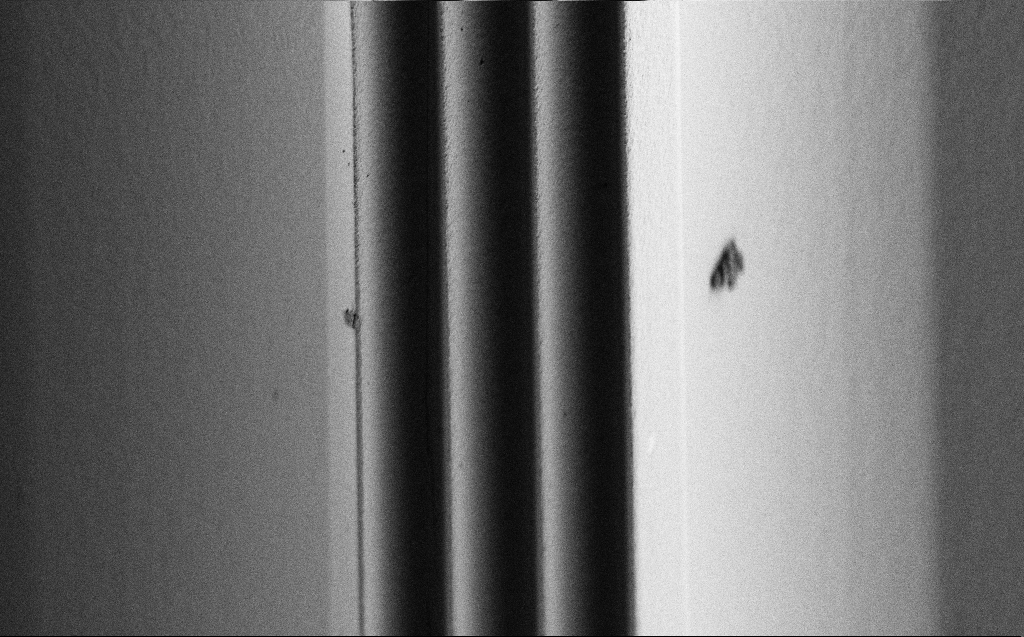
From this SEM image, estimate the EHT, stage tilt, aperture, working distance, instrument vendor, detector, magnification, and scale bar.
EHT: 1 kV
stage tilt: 44.6°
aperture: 30 µm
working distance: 5 mm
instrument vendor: Zeiss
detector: SE2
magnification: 2.94 K X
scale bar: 20000 nm